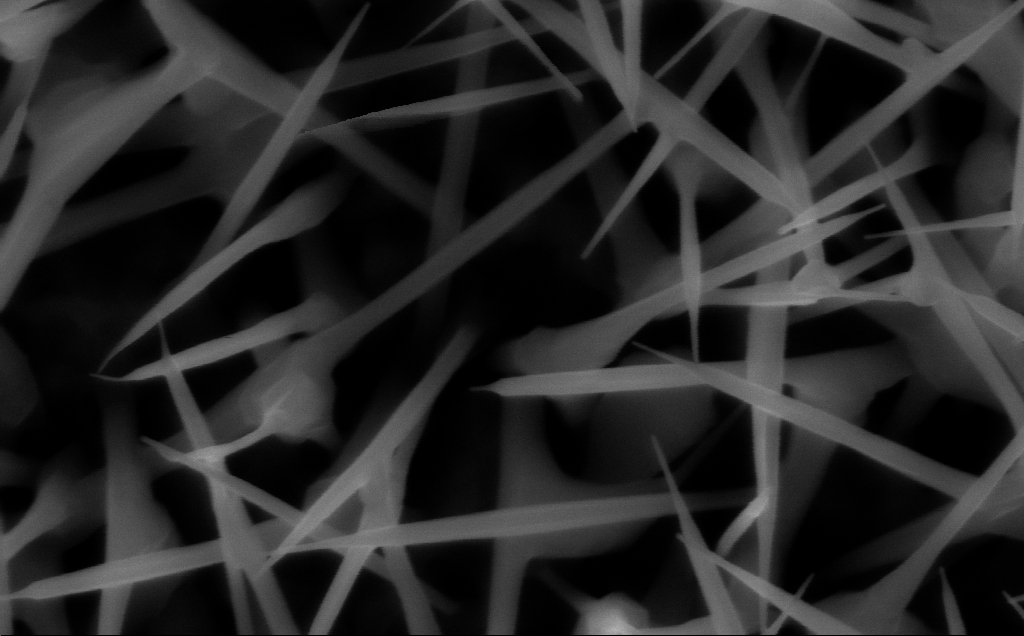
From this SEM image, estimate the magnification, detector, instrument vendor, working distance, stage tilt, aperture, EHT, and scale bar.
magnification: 150 K X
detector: InLens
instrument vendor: Zeiss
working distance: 7 mm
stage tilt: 0°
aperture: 30 µm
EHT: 10 kV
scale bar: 100 nm